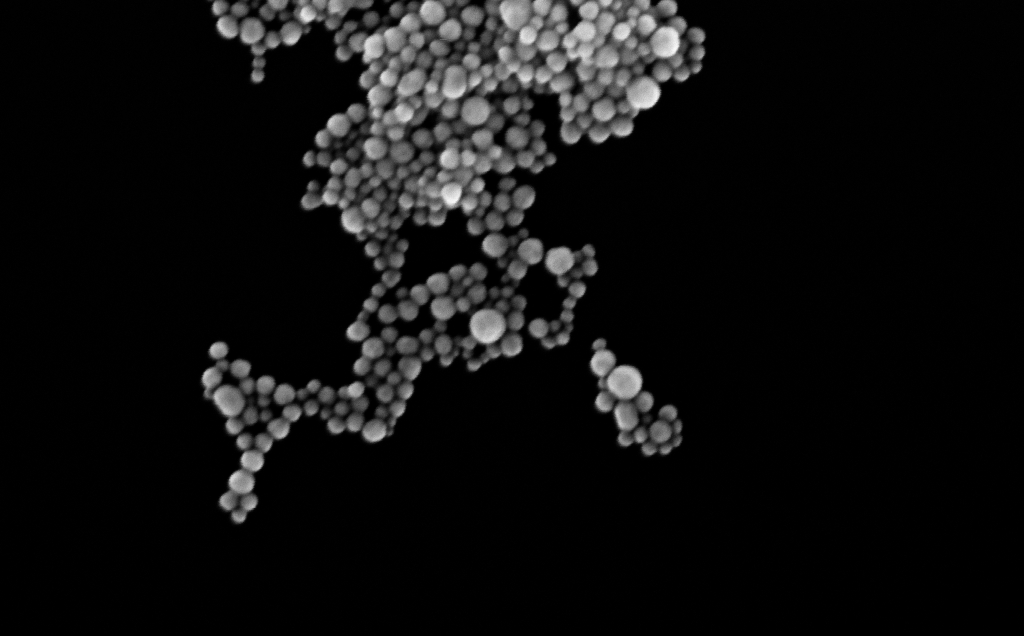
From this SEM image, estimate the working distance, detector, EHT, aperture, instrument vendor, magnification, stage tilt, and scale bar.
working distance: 3 mm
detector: InLens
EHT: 10 kV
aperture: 30 µm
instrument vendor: Zeiss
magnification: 266.24 K X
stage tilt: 0°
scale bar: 200 nm